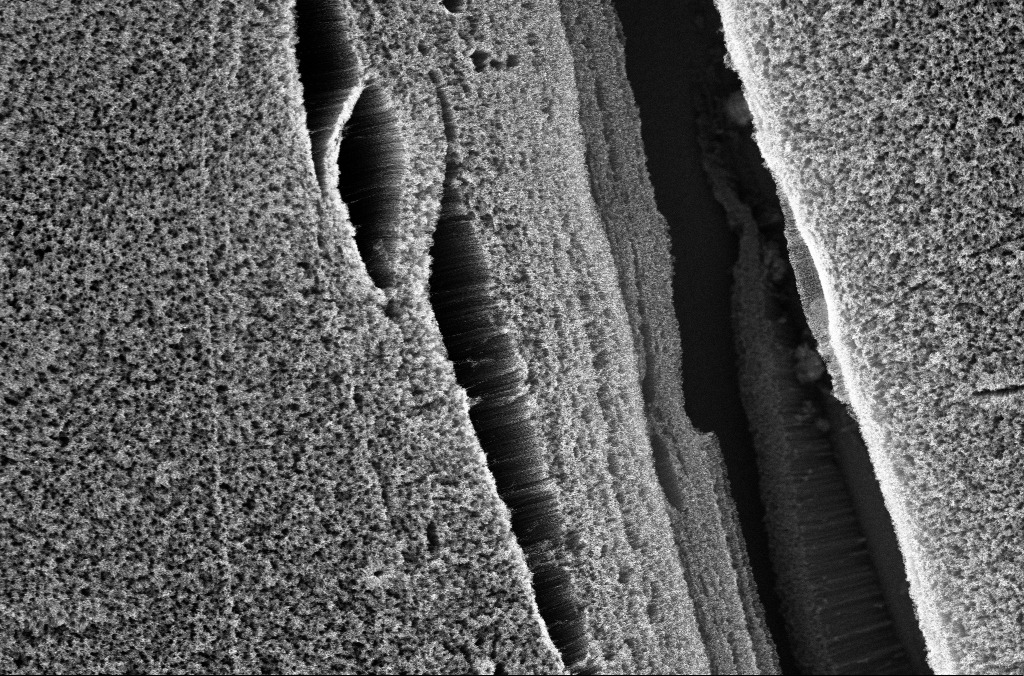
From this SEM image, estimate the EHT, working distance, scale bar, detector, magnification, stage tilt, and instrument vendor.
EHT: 5 kV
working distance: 3.9 mm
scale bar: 20000 nm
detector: InLens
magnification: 1.5 K X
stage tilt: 0°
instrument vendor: Zeiss